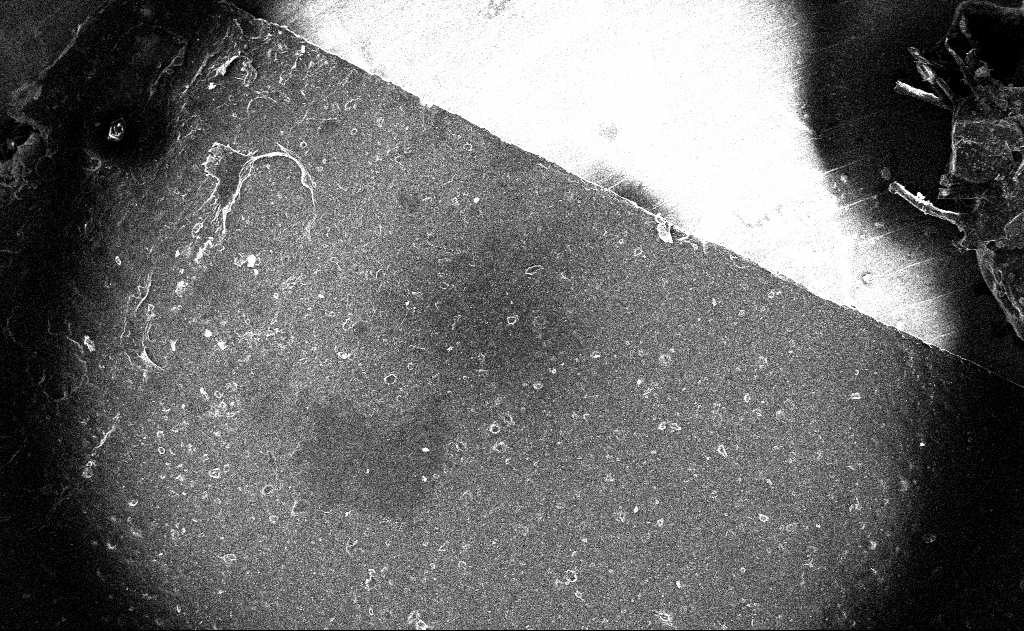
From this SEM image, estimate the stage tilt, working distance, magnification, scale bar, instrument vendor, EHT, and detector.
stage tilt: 0°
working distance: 3 mm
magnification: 0.122 K X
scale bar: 100000 nm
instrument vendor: Zeiss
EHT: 10 kV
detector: InLens